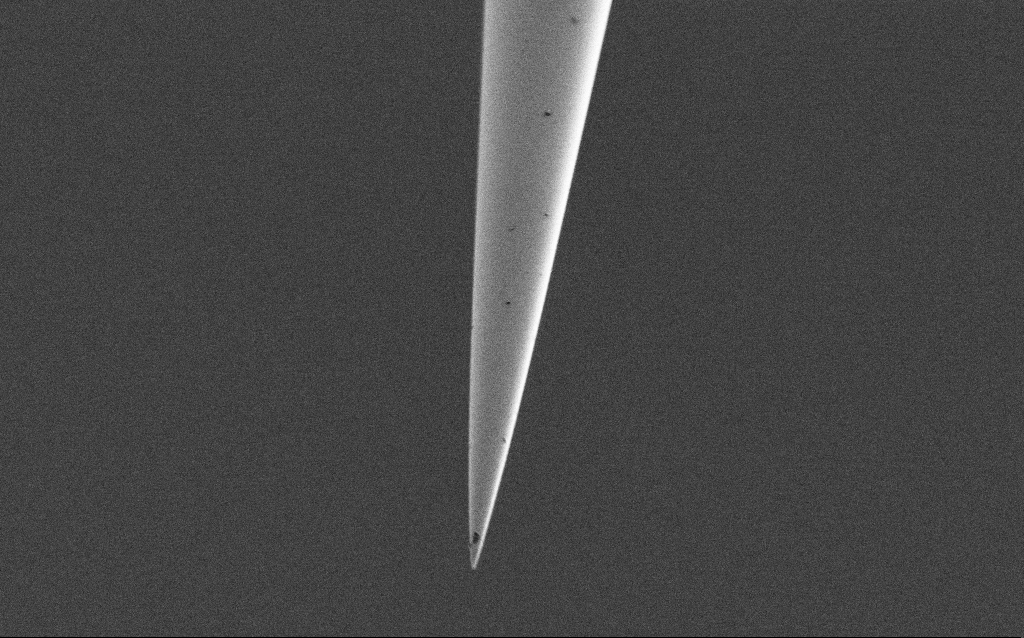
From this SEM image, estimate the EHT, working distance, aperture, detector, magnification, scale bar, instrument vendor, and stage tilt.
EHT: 1 kV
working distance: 6.9 mm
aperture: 30 µm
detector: SE2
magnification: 10 K X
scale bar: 2000 nm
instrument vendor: Zeiss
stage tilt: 45°